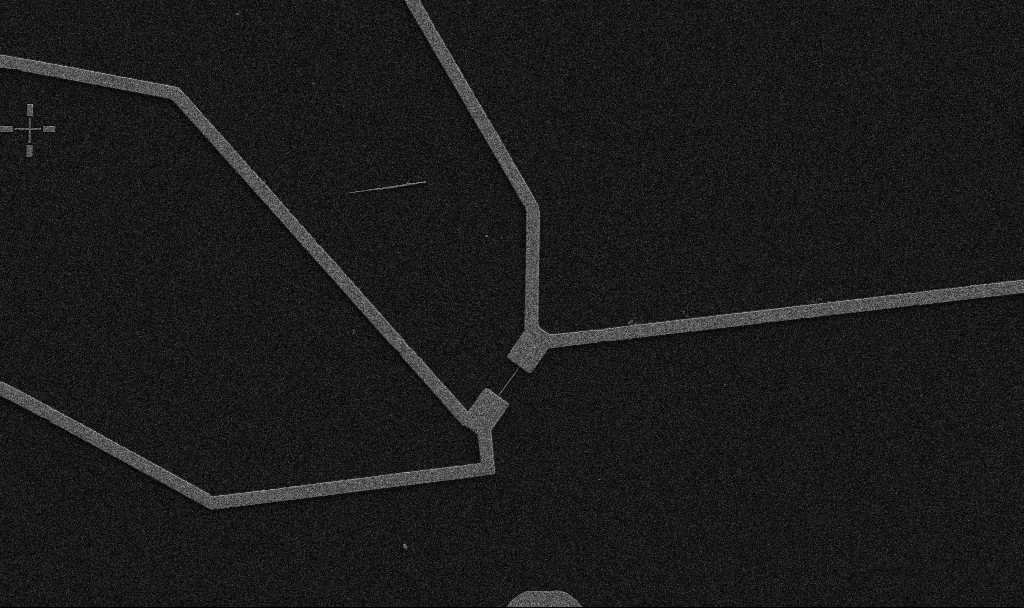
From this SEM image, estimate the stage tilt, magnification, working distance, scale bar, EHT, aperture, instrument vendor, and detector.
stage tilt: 0°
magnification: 5 K X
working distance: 10.7 mm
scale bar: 10000 nm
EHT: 5 kV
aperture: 30 µm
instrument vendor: Zeiss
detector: SE2